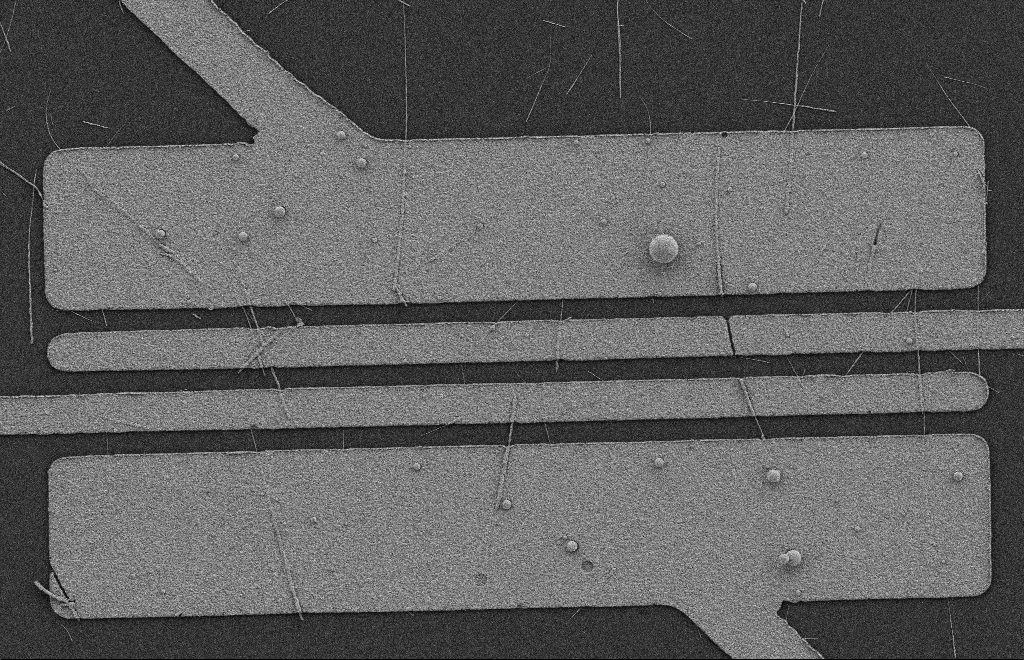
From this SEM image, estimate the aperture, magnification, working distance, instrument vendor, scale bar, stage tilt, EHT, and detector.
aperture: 20 µm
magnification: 5.65 K X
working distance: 9 mm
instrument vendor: Zeiss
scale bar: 2000 nm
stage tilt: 0°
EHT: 2 kV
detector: SE2